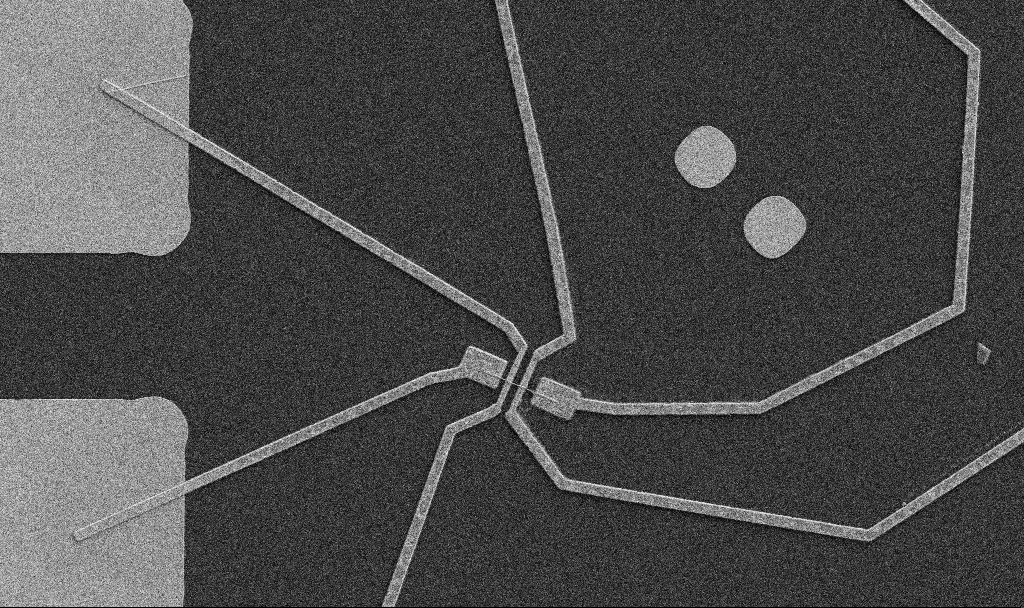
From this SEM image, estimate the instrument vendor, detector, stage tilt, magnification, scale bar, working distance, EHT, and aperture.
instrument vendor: Zeiss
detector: SE2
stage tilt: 0°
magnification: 5 K X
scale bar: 10000 nm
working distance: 8.7 mm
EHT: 5 kV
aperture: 30 µm